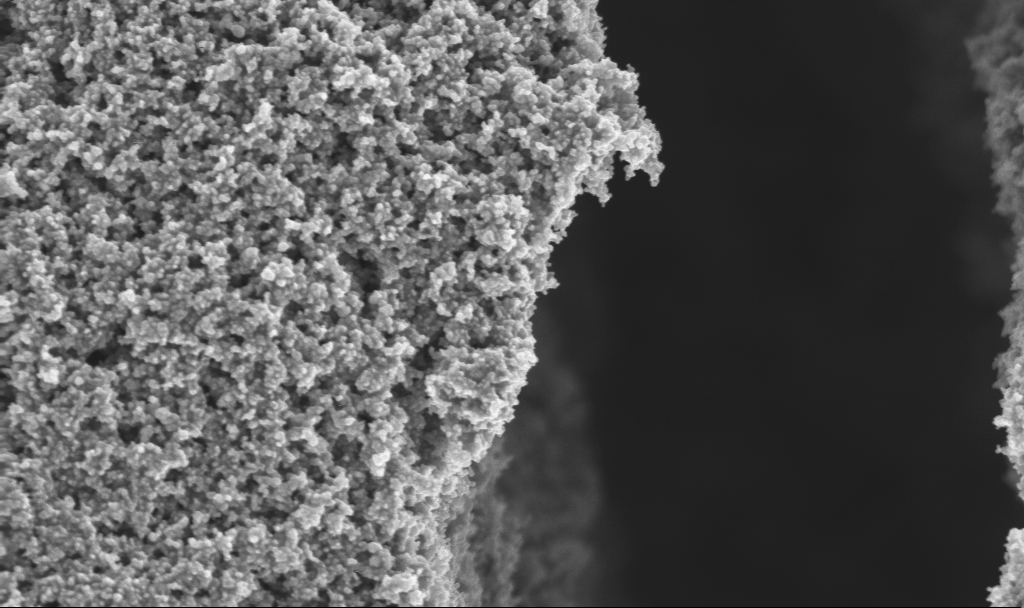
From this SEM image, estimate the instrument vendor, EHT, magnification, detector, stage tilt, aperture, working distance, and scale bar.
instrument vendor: Zeiss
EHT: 3 kV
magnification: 55.95 K X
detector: InLens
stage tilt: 0°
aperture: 30 µm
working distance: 2.4 mm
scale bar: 1000 nm